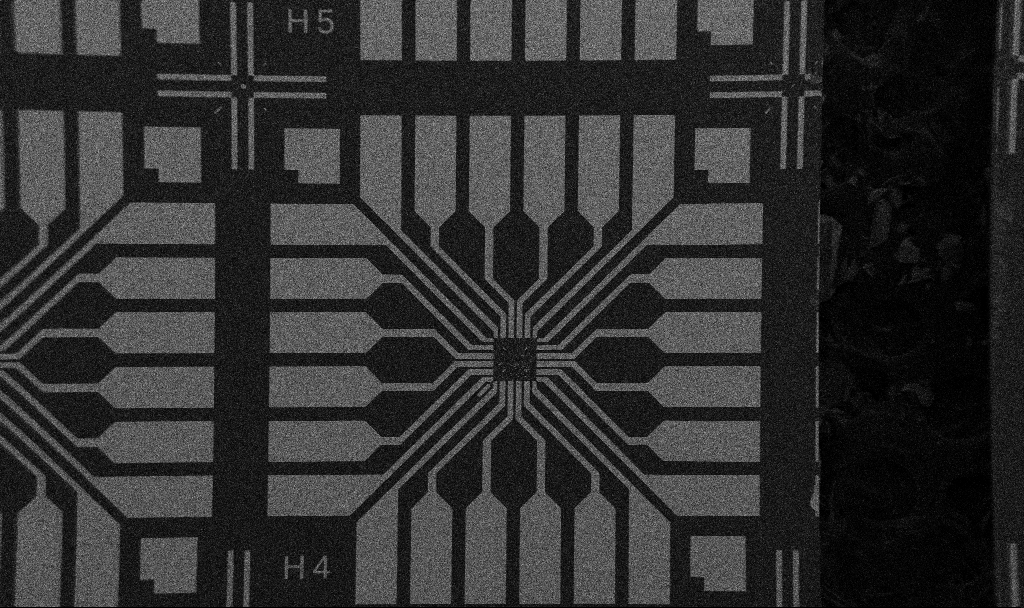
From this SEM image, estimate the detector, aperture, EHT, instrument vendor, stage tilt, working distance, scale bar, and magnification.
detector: SE2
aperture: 30 µm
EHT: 5 kV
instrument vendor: Zeiss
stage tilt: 0°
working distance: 10.7 mm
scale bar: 200000 nm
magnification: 0.1 K X